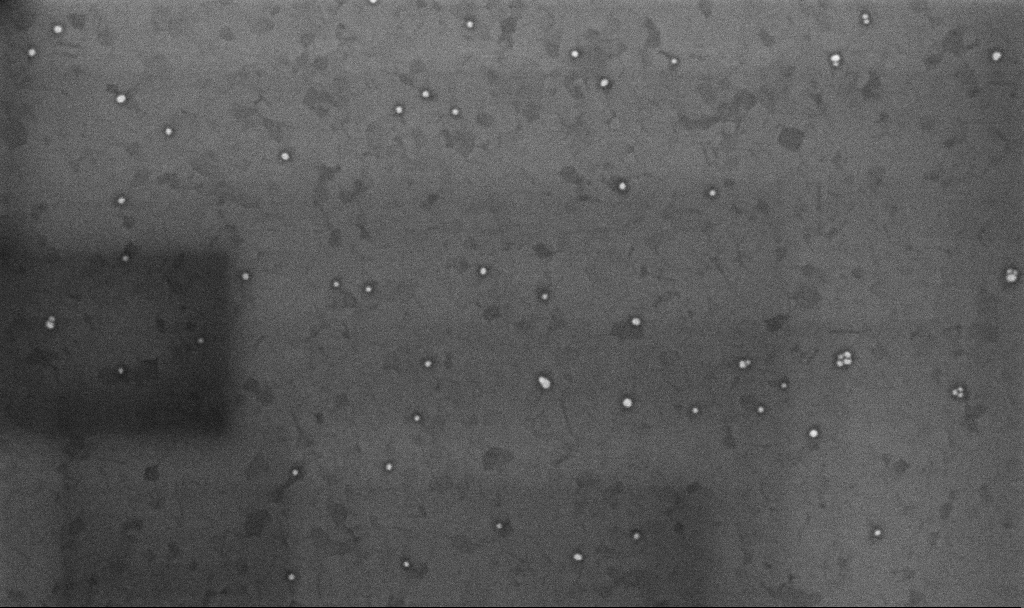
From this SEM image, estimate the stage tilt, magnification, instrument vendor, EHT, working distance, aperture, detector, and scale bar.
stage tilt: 0°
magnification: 100.38 K X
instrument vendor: Zeiss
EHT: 10 kV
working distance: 3.3 mm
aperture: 30 µm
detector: InLens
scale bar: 200 nm